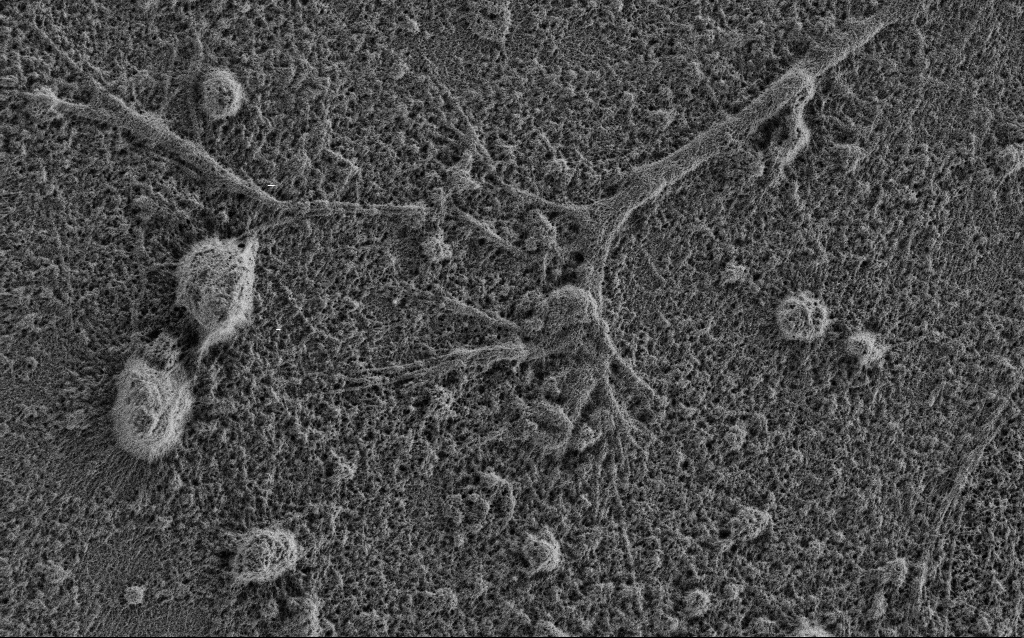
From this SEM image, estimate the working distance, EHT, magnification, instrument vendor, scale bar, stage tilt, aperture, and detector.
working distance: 3.4 mm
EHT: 0.9 kV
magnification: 10 K X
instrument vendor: Zeiss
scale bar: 2000 nm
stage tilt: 0°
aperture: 30 µm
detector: SE2